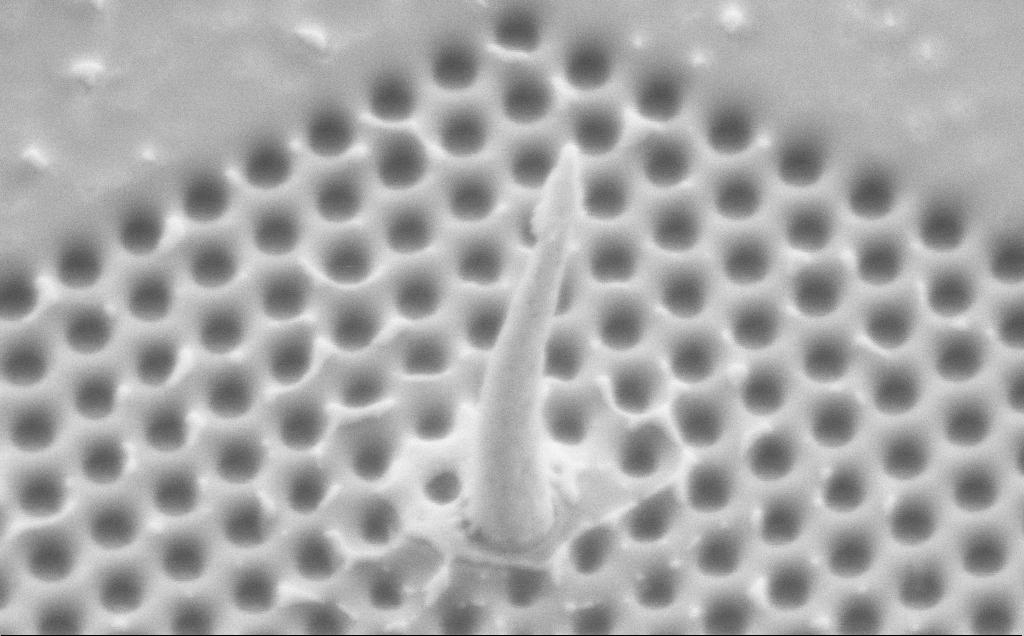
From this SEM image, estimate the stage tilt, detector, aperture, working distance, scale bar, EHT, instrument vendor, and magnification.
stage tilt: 34.3°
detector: SE2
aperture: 30 µm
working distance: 6 mm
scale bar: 200 nm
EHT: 3 kV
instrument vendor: Zeiss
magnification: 109.14 K X